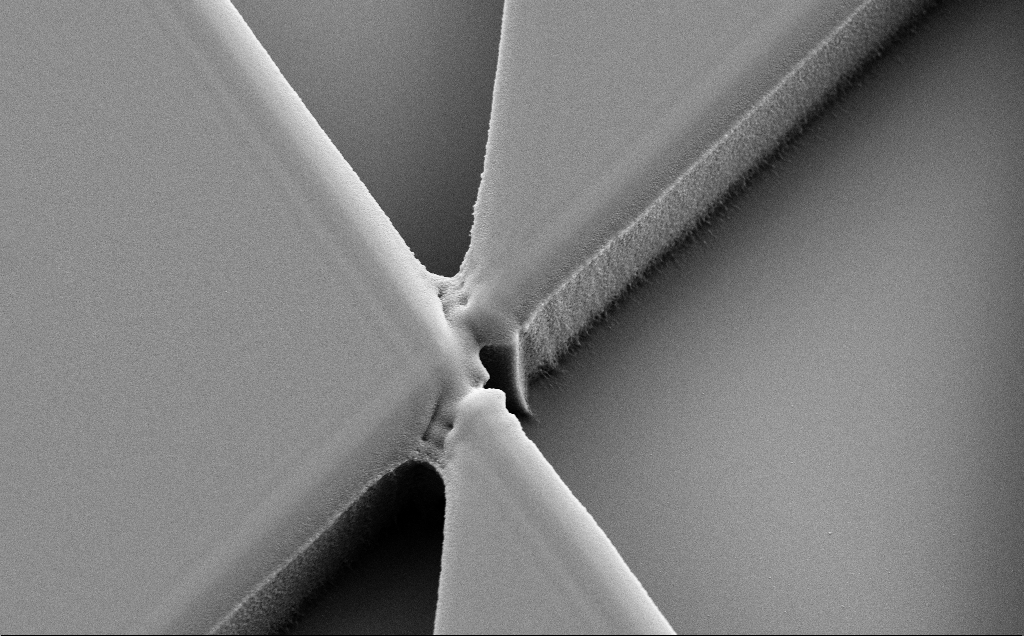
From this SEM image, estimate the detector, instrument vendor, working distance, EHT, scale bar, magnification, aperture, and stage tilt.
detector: SE2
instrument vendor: Zeiss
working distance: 8 mm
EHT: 5 kV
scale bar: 10000 nm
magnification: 6.19 K X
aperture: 30 µm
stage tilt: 30°